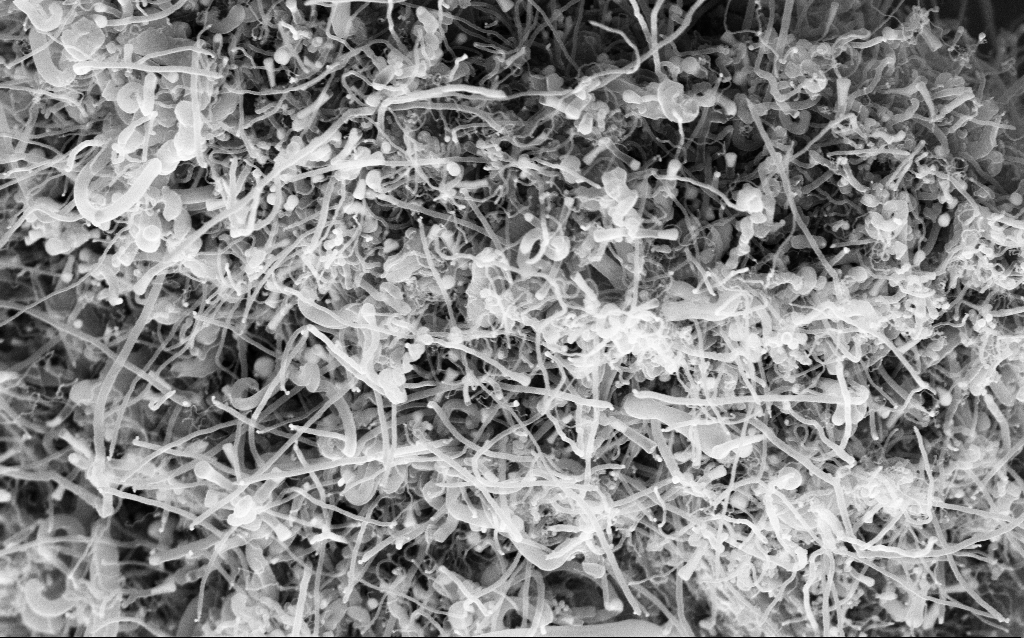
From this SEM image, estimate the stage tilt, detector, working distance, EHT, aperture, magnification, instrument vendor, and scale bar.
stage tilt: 45°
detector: InLens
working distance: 6.6 mm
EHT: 5 kV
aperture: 30 µm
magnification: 39.97 K X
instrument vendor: Zeiss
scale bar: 1000 nm